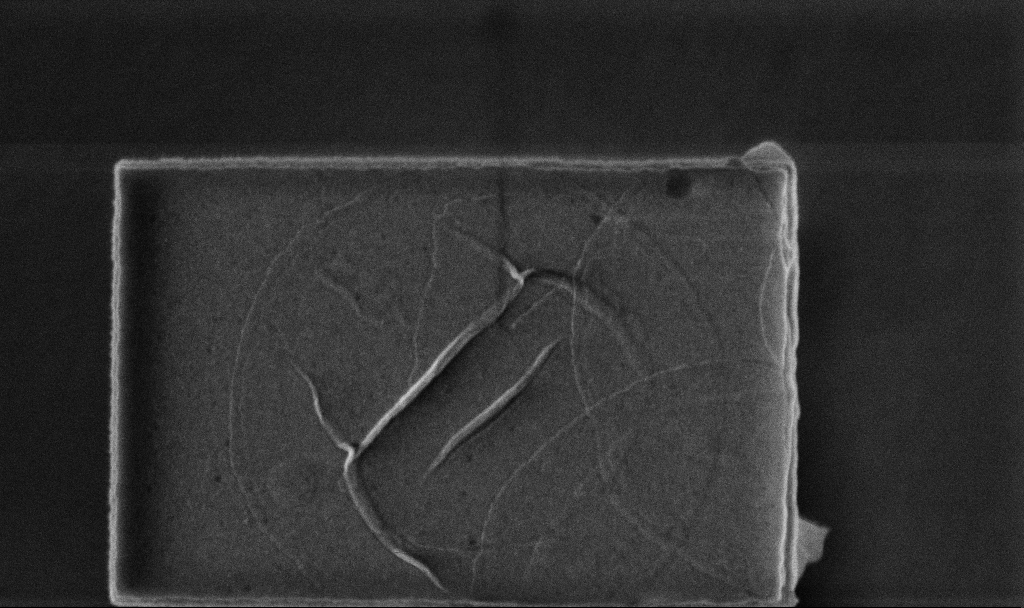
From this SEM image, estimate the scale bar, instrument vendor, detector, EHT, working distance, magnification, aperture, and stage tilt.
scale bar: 1000 nm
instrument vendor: Zeiss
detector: InLens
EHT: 5 kV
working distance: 3.2 mm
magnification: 55.14 K X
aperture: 30 µm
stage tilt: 0°